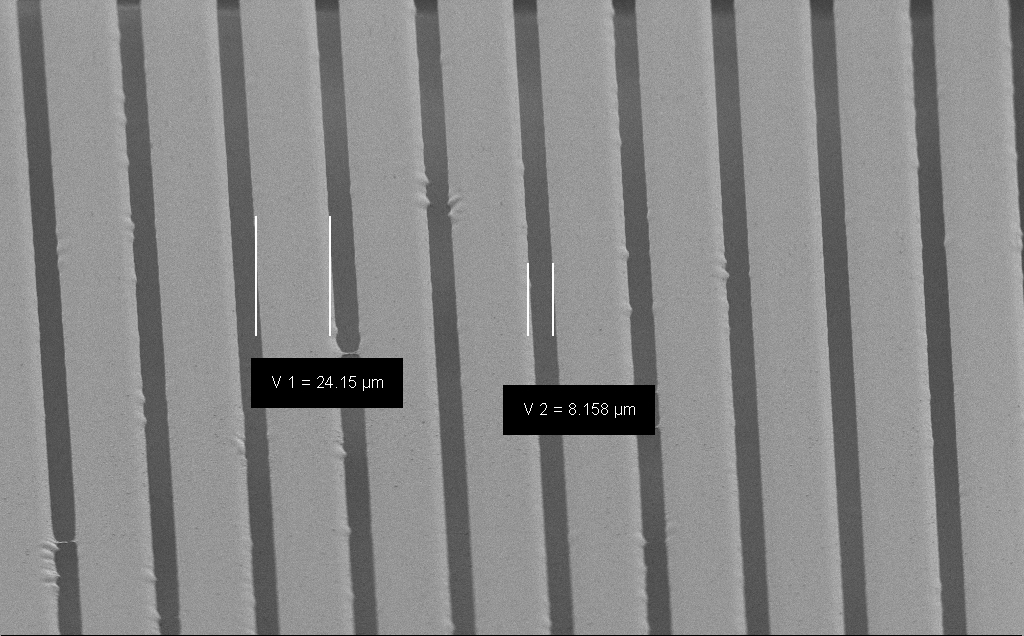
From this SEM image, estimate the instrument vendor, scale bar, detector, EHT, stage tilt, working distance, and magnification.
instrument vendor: Zeiss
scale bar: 20000 nm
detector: SE2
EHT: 1.2 kV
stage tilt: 45°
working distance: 7 mm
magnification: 1.13 K X